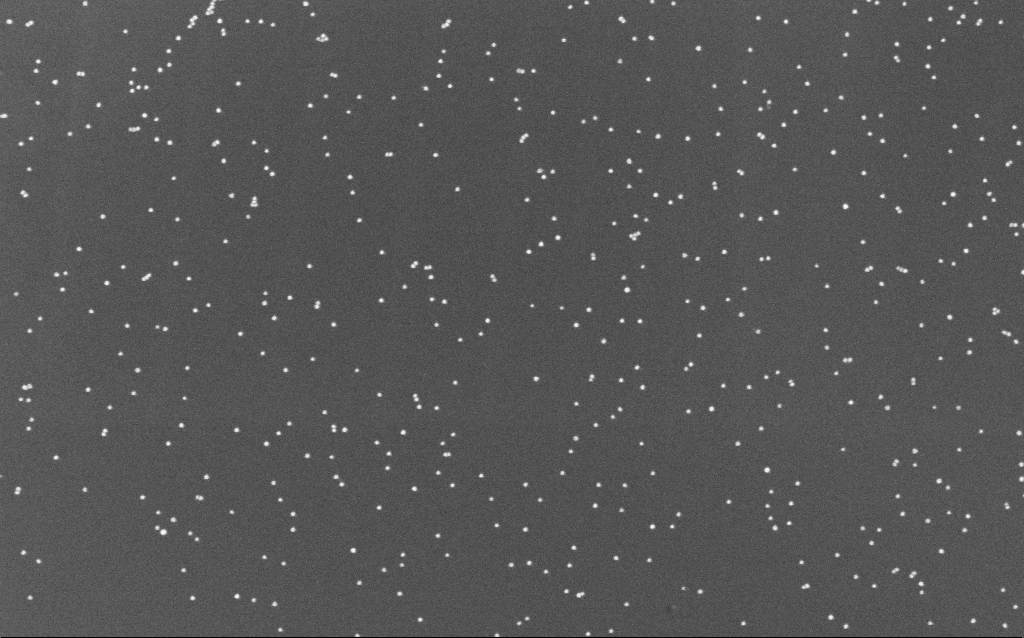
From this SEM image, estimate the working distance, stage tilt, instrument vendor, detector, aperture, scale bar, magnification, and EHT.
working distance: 6.6 mm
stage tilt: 0°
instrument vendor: Zeiss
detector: InLens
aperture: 30 µm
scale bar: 200 nm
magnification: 100 K X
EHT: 10 kV